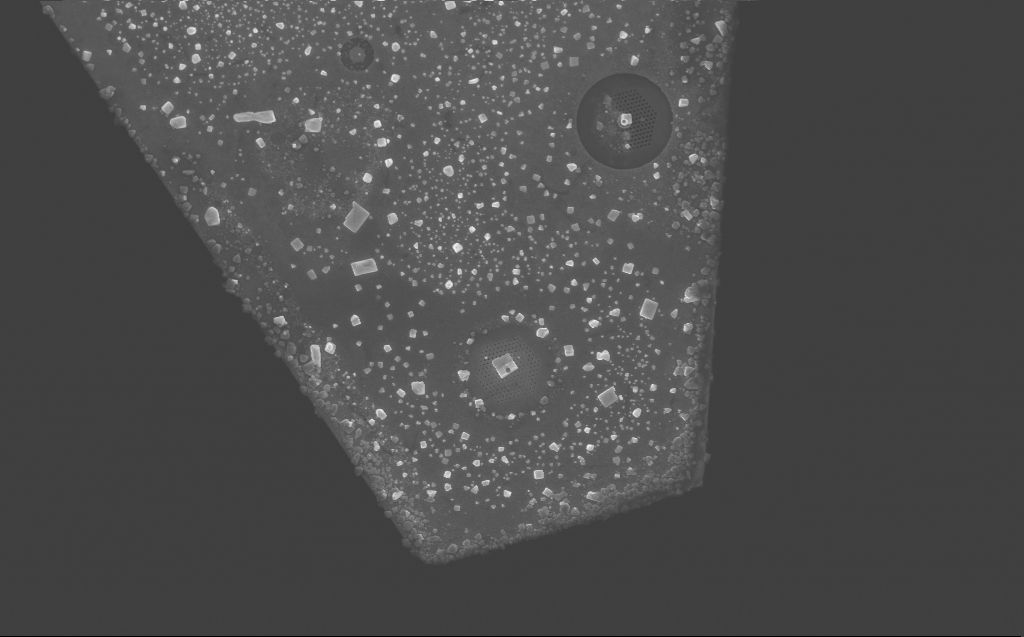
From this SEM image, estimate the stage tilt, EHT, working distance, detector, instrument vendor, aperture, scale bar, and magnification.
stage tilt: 0°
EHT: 10 kV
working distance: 6 mm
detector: InLens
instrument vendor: Zeiss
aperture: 30 µm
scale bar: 10000 nm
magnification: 5.94 K X